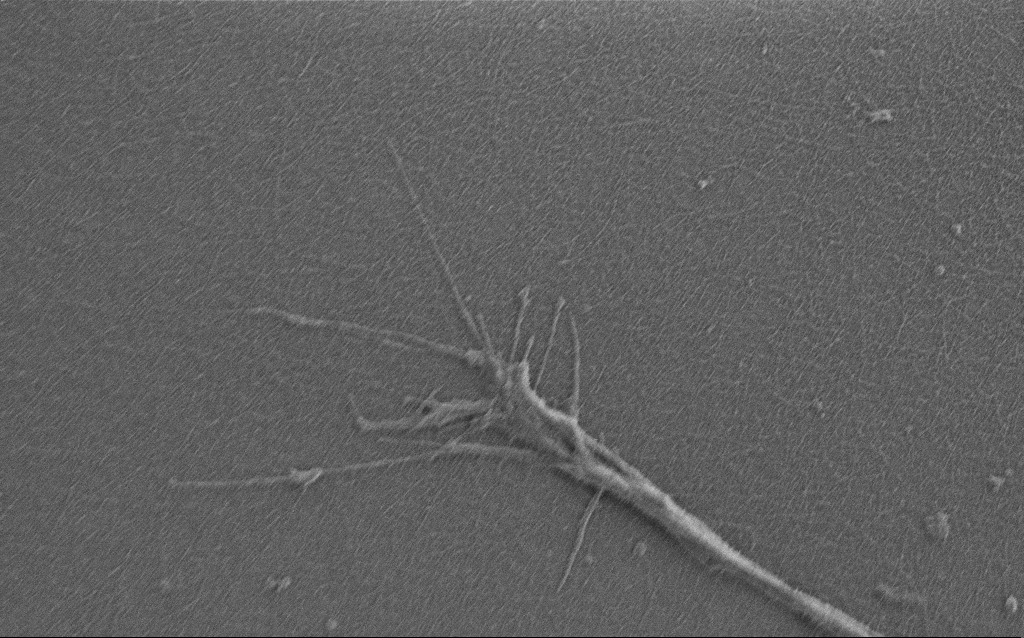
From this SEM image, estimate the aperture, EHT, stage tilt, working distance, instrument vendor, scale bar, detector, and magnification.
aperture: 30 µm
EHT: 0.9 kV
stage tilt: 0°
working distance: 6 mm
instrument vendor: Zeiss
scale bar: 2000 nm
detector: SE2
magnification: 10 K X